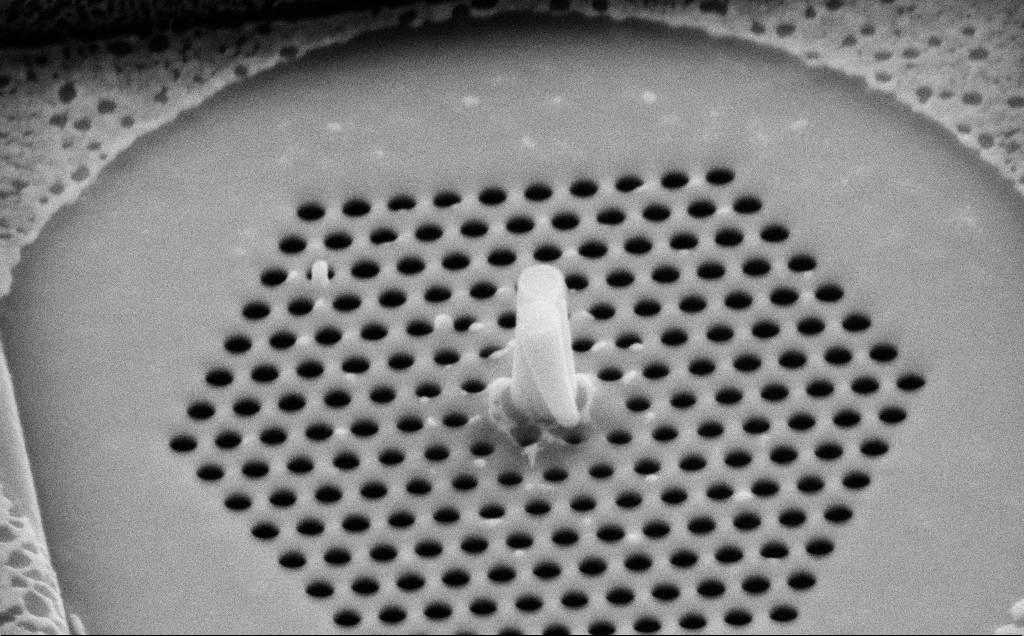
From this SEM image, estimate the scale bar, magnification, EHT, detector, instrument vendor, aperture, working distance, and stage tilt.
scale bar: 1000 nm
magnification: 67.83 K X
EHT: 10 kV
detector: SE2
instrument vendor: Zeiss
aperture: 30 µm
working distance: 8 mm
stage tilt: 45°